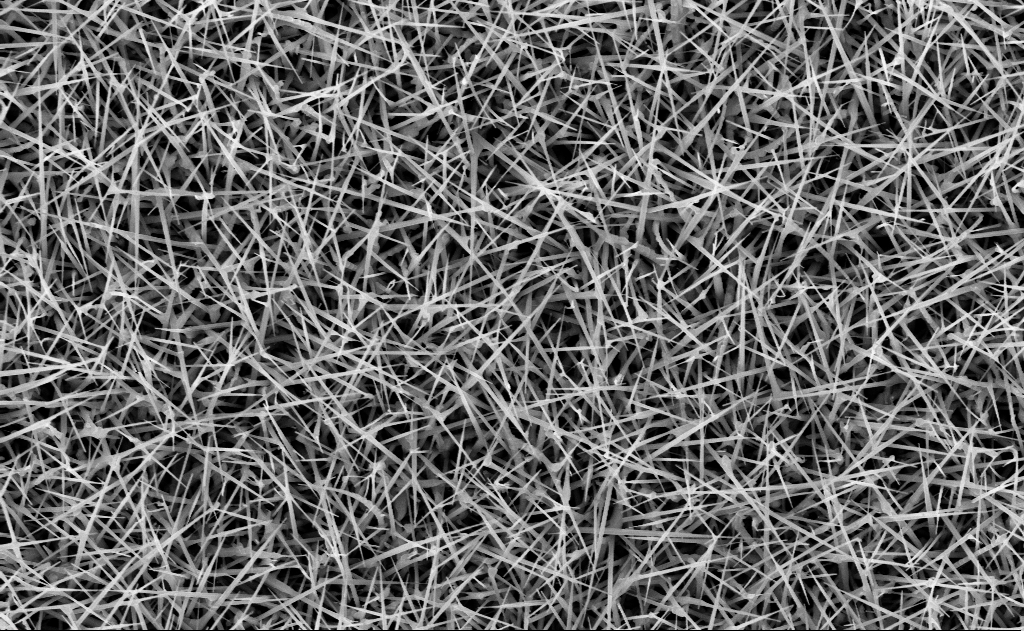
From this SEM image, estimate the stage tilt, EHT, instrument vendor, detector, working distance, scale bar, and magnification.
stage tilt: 0°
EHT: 10 kV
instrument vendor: Zeiss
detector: InLens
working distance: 15 mm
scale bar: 2000 nm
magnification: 20 K X